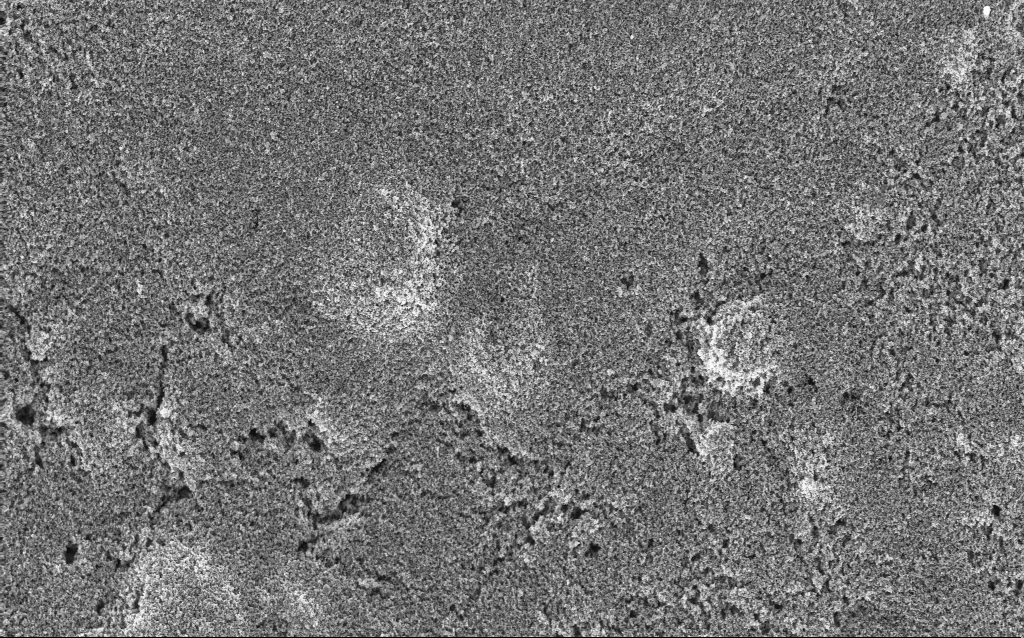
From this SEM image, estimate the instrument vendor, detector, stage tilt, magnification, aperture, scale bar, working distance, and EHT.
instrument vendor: Zeiss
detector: InLens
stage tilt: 0°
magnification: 15.33 K X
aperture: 30 µm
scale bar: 1000 nm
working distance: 2.7 mm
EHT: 5 kV